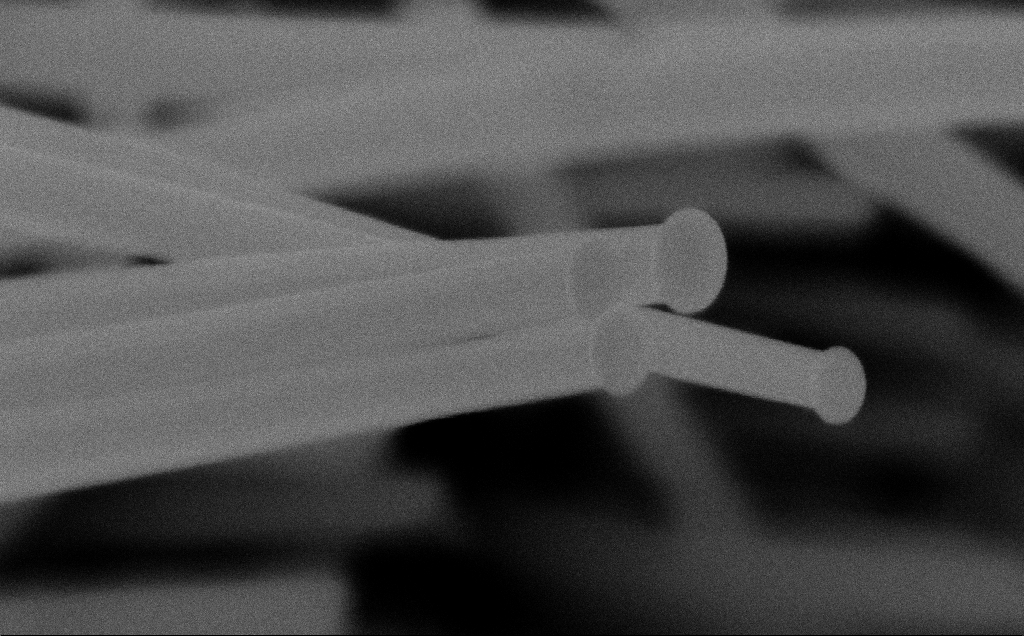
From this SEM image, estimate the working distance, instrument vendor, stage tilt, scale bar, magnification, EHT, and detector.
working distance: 6 mm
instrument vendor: Zeiss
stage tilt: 0°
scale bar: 200 nm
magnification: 242.77 K X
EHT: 10 kV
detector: InLens